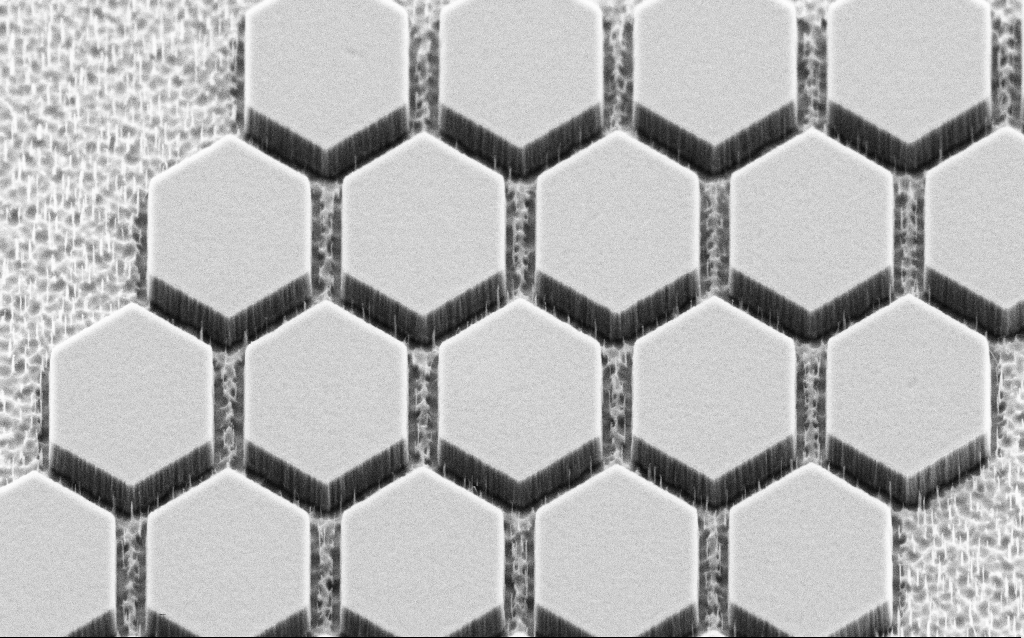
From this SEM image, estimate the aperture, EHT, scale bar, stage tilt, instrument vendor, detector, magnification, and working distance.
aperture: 30 µm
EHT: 3 kV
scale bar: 1000 nm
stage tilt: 45°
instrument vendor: Zeiss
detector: SE2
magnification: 22.88 K X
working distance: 8 mm